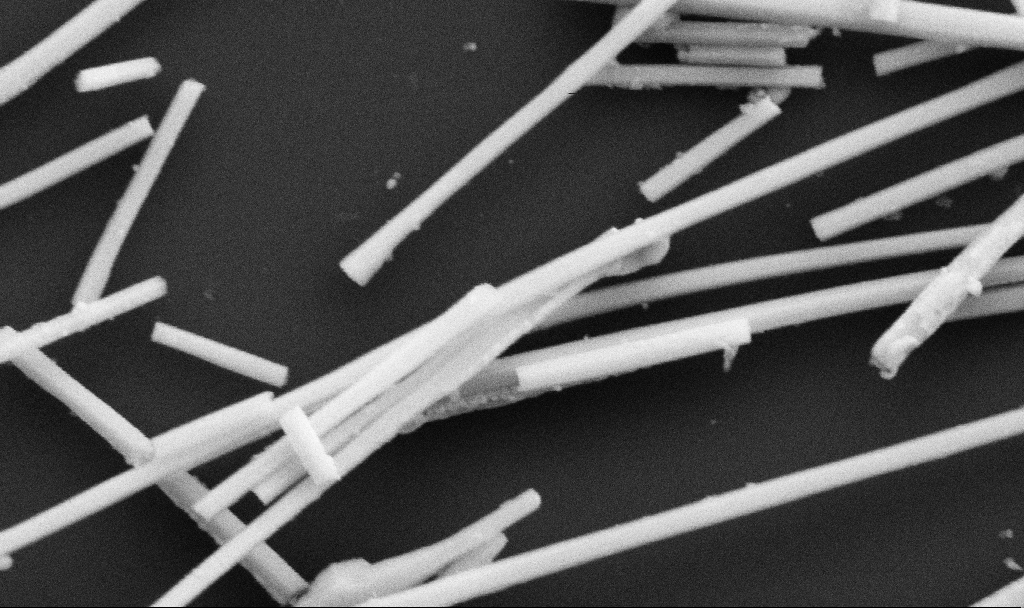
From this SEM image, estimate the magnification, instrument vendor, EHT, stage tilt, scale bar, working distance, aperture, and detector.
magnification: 93.5 K X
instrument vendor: Zeiss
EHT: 5 kV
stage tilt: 0°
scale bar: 200 nm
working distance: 10.7 mm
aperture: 30 µm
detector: SE2